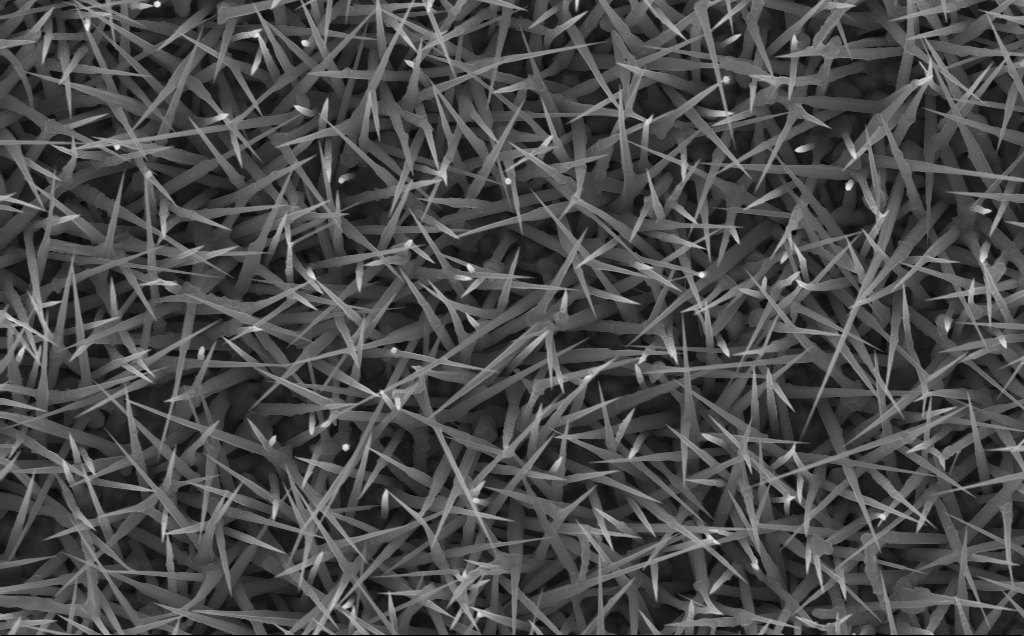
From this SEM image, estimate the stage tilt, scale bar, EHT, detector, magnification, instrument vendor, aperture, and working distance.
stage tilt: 0°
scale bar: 2000 nm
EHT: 10 kV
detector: InLens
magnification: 20 K X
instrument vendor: Zeiss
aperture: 30 µm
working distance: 4 mm